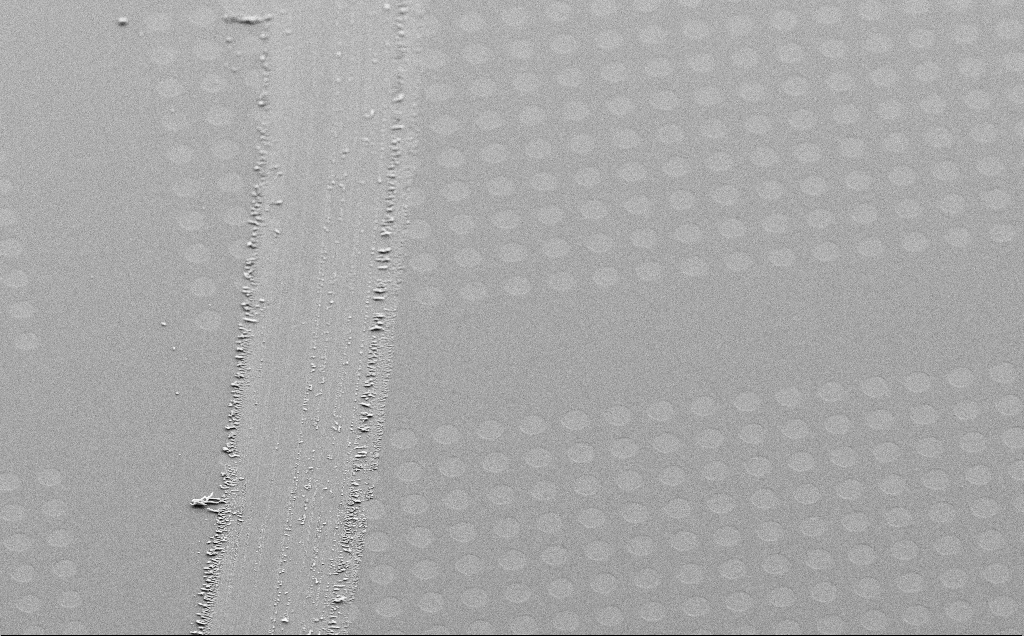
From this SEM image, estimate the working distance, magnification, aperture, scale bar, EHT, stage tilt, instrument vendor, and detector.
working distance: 6 mm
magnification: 0.517 K X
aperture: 30 µm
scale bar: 100000 nm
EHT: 10 kV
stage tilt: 40.4°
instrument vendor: Zeiss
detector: SE2